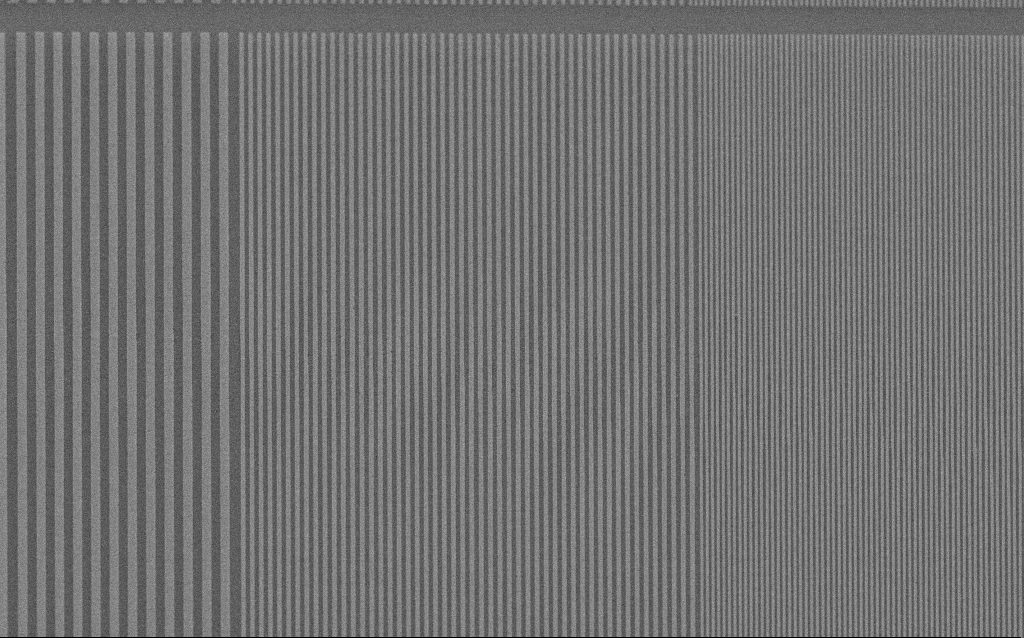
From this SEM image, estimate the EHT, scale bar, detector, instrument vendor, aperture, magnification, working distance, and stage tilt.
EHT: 20 kV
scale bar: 10000 nm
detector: SE2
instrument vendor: Zeiss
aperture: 30 µm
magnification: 3.34 K X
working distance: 6.7 mm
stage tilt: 45°